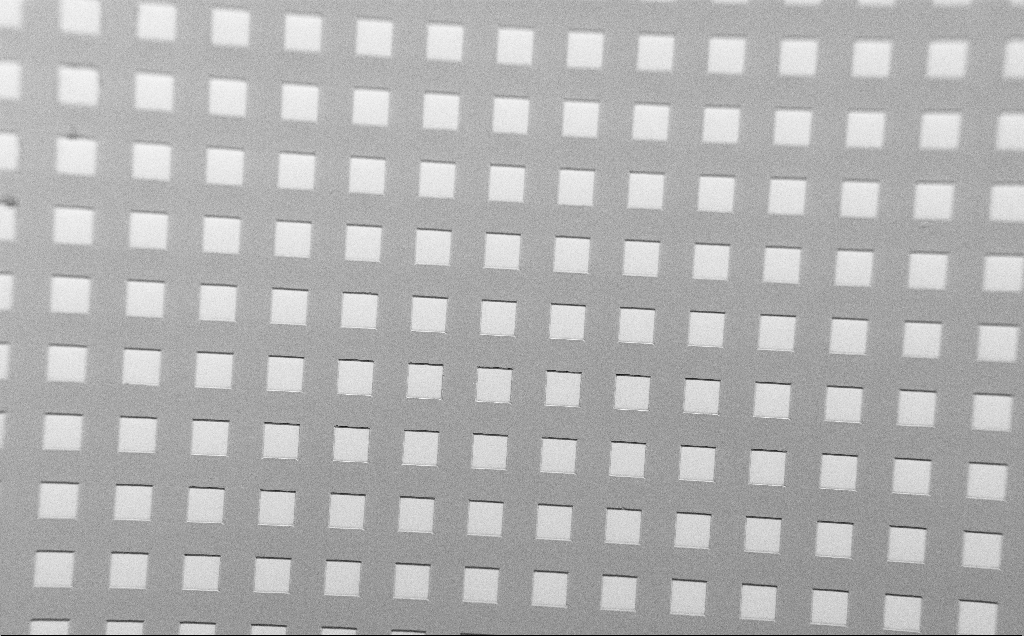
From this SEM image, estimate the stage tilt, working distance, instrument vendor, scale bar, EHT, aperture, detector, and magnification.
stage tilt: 45°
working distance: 7 mm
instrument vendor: Zeiss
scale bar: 200000 nm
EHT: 1.5 kV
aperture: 30 µm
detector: SE2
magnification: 0.255 K X